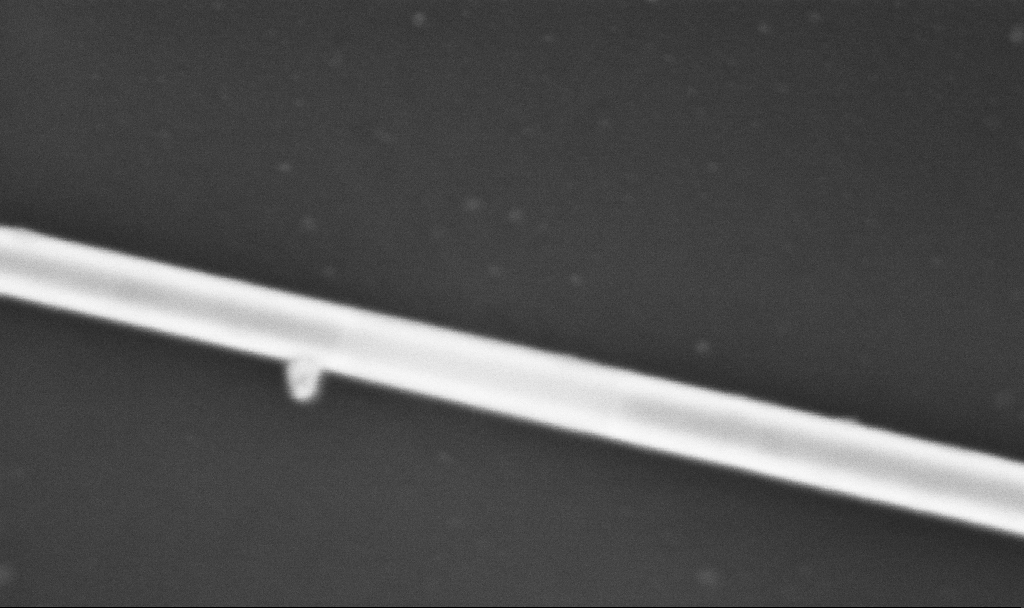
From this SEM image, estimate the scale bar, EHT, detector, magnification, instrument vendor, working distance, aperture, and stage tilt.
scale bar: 200 nm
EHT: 5 kV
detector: InLens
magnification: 300 K X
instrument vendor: Zeiss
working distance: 6.7 mm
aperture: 30 µm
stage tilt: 0°